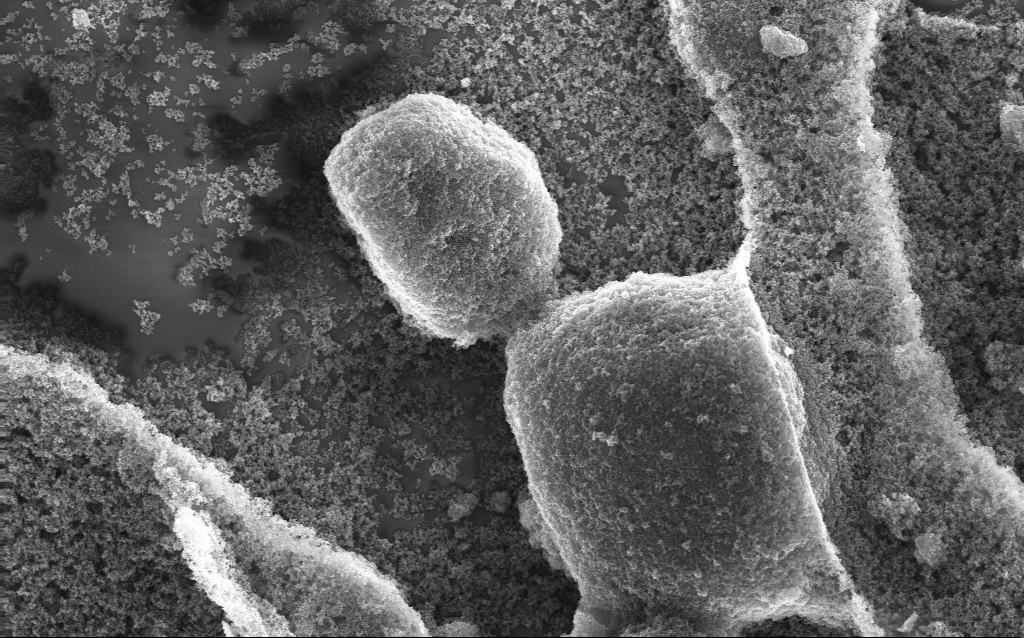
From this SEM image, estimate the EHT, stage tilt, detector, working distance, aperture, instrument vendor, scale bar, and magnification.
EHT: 10 kV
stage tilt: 0°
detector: InLens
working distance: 2.4 mm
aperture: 30 µm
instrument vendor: Zeiss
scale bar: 1000 nm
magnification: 20.87 K X